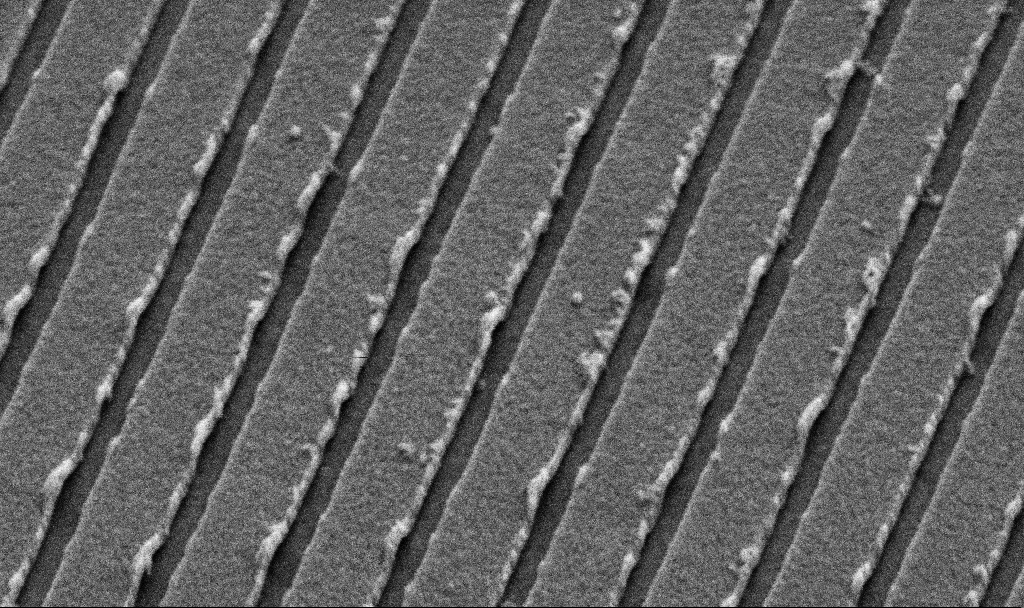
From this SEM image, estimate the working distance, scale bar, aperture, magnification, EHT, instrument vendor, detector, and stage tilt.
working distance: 10.6 mm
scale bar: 1000 nm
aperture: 30 µm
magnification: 67.66 K X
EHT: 5 kV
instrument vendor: Zeiss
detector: SE2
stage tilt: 45°